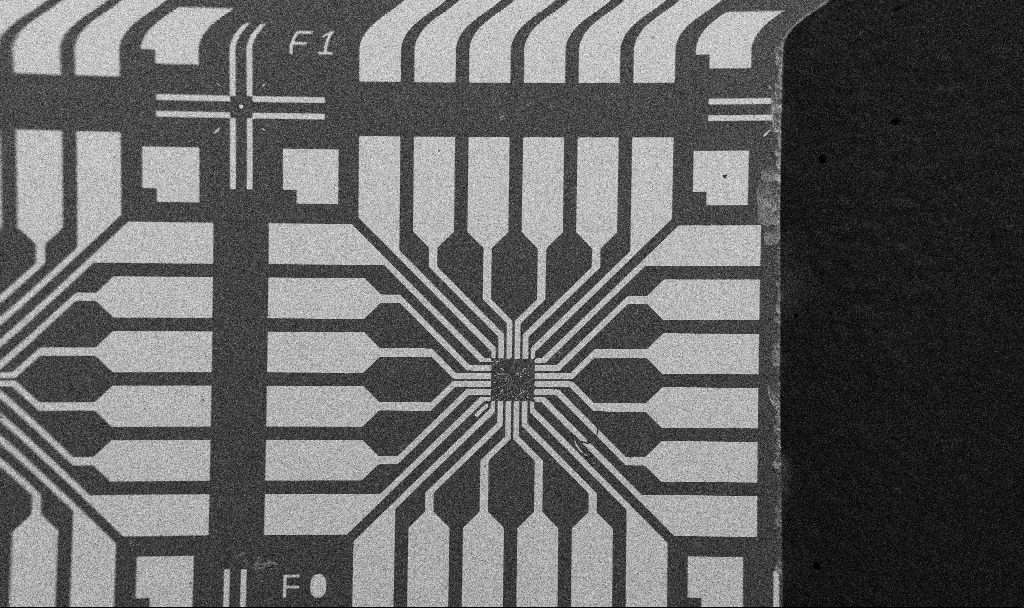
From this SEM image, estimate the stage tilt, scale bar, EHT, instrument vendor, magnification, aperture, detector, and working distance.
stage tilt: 0°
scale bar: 200000 nm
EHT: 5 kV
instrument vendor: Zeiss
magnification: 0.1 K X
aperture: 30 µm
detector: SE2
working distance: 10.7 mm